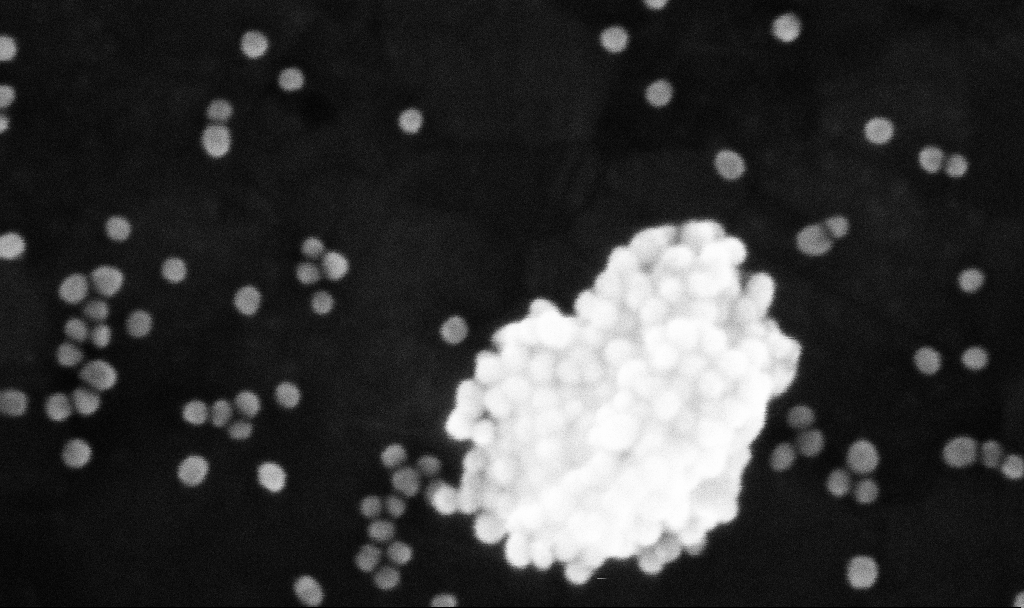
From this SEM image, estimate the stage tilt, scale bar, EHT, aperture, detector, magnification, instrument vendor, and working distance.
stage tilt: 0°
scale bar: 100 nm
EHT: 10 kV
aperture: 30 µm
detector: InLens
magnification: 608.57 K X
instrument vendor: Zeiss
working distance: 3.7 mm